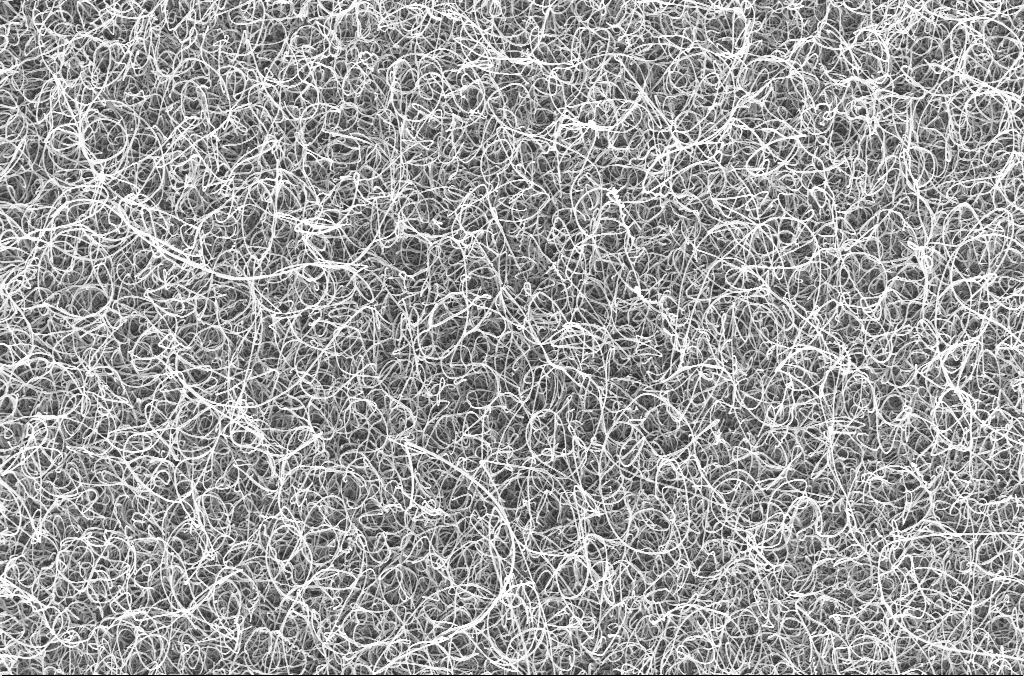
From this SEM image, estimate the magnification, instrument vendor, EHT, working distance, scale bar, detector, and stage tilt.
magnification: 15 K X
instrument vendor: Zeiss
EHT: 20 kV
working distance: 4.5 mm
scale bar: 1000 nm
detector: InLens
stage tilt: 0°